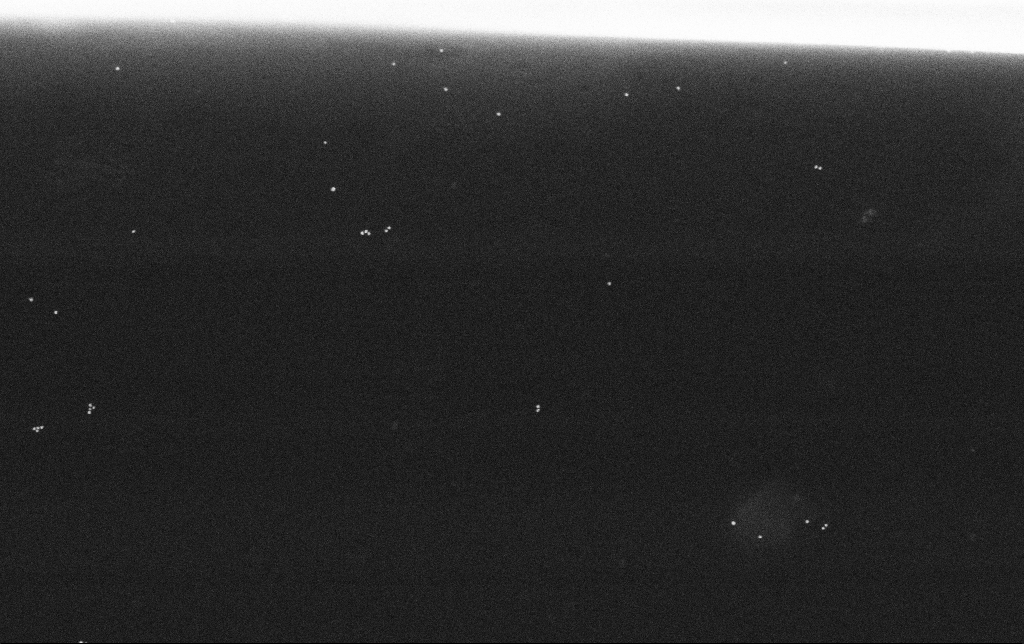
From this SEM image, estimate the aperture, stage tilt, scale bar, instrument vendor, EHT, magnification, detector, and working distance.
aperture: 30 µm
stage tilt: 0°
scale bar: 200 nm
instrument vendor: Zeiss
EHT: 30 kV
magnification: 100 K X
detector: SE2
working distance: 10.8 mm